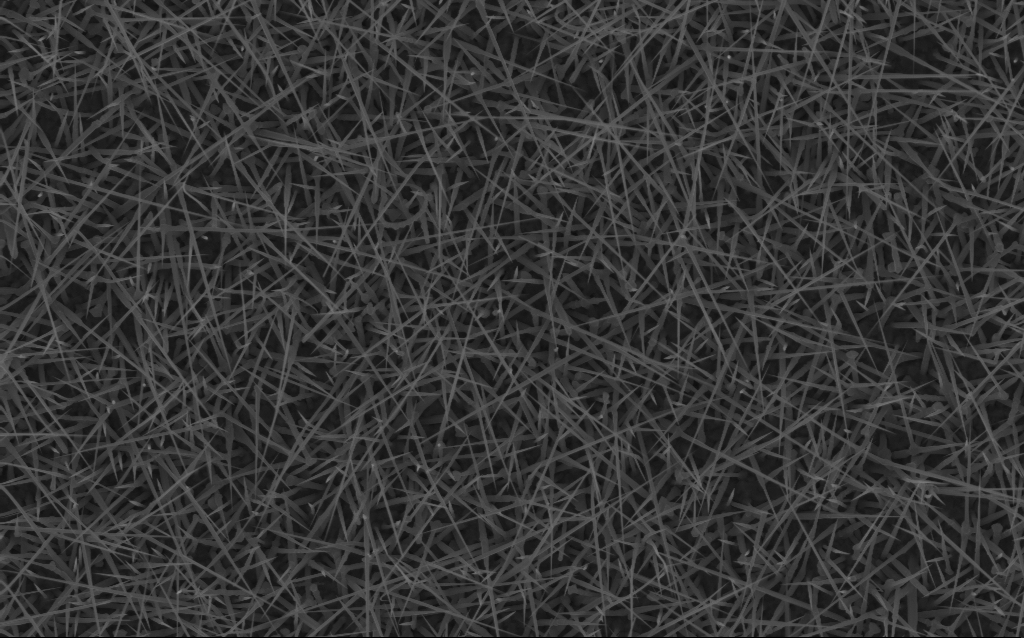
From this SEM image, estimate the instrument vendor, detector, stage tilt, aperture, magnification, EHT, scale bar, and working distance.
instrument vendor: Zeiss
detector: InLens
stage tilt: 0°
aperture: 30 µm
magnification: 10 K X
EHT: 10 kV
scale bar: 2000 nm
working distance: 7 mm